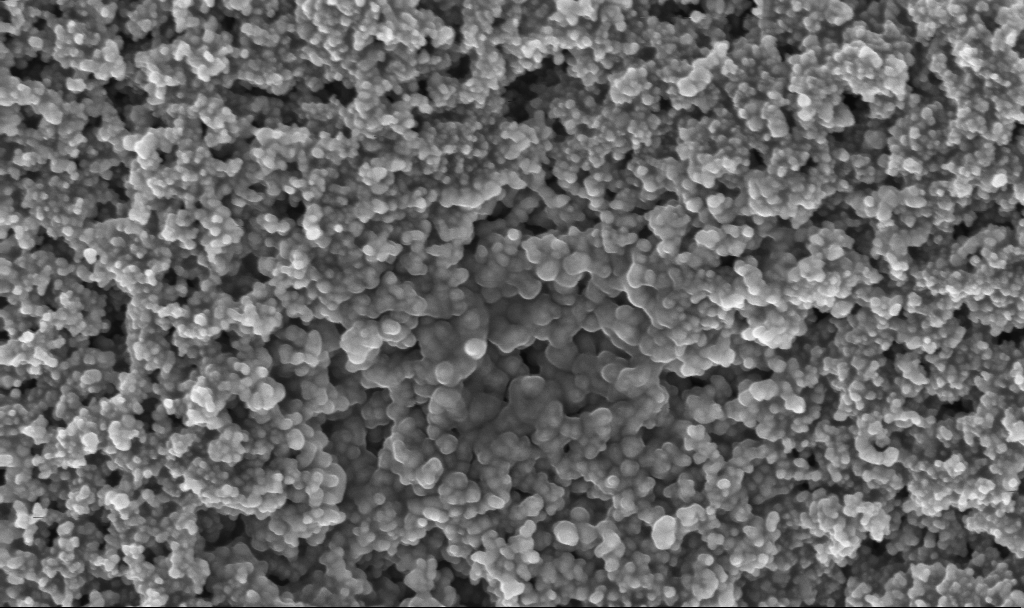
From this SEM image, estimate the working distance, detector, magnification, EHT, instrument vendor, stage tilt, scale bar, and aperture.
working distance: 2.4 mm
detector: InLens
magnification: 100 K X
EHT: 3 kV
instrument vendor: Zeiss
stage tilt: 0°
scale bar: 200 nm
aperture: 30 µm